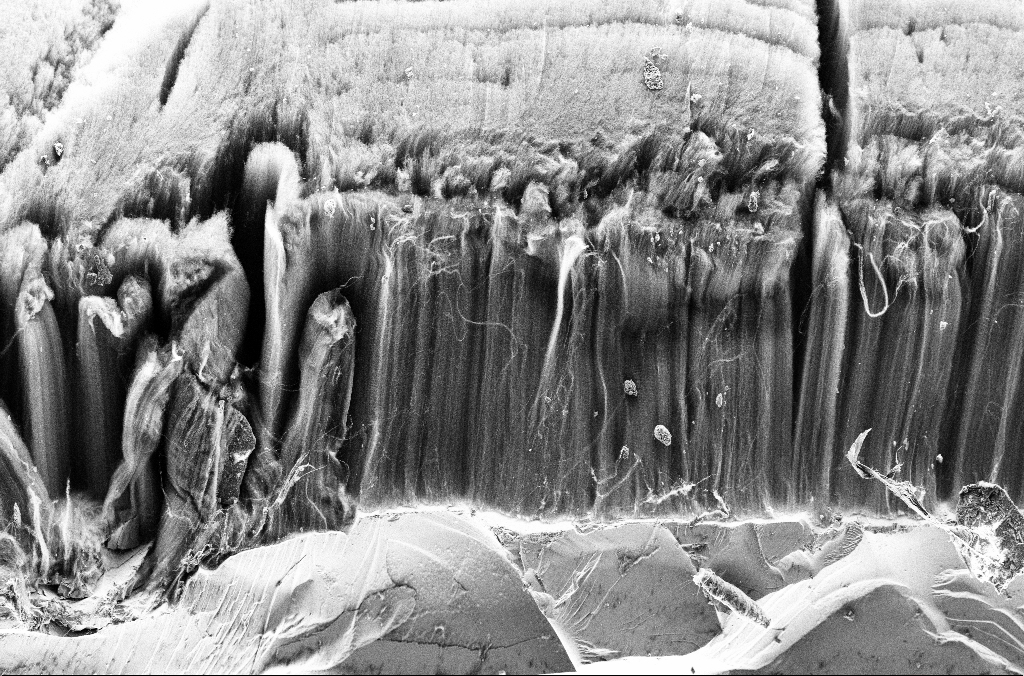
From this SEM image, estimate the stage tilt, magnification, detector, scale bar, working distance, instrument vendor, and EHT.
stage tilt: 45°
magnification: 1 K X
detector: SE2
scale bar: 20000 nm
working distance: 3.4 mm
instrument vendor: Zeiss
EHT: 3 kV